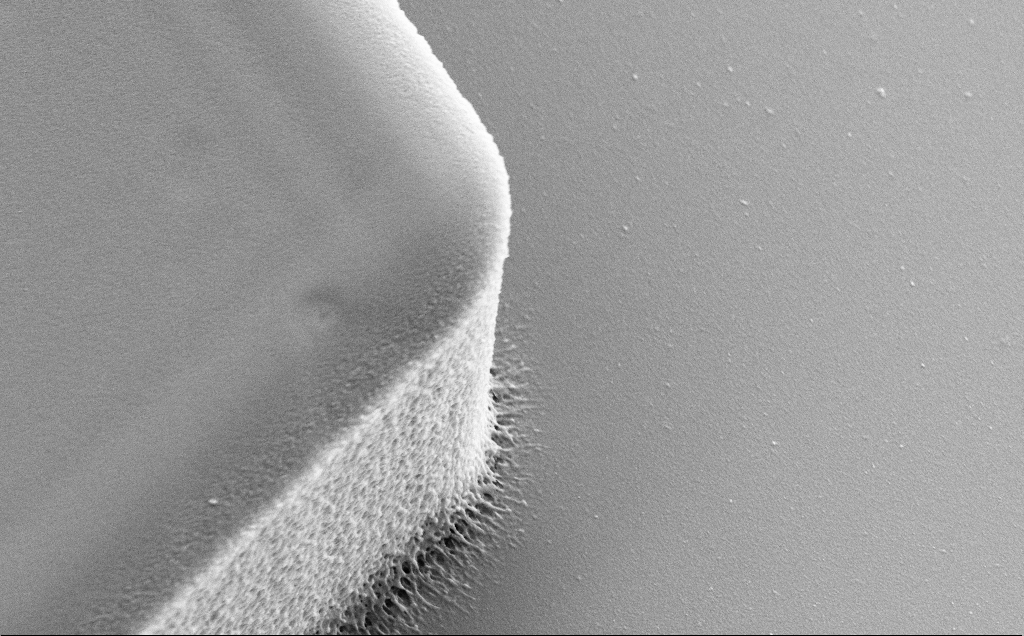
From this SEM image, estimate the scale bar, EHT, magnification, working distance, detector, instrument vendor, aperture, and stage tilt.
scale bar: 1000 nm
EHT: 5 kV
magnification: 18.07 K X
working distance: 8 mm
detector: SE2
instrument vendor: Zeiss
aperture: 30 µm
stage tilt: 30°